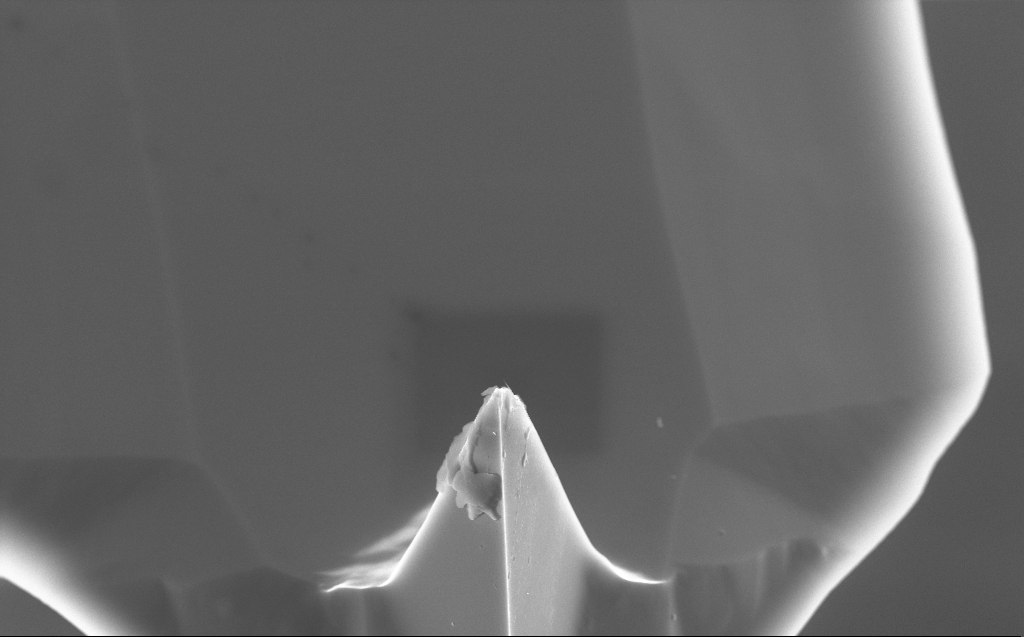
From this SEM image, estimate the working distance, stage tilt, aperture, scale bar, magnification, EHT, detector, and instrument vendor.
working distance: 3 mm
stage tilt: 45°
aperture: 30 µm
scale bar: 2000 nm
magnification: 10.26 K X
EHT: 10 kV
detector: InLens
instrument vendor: Zeiss